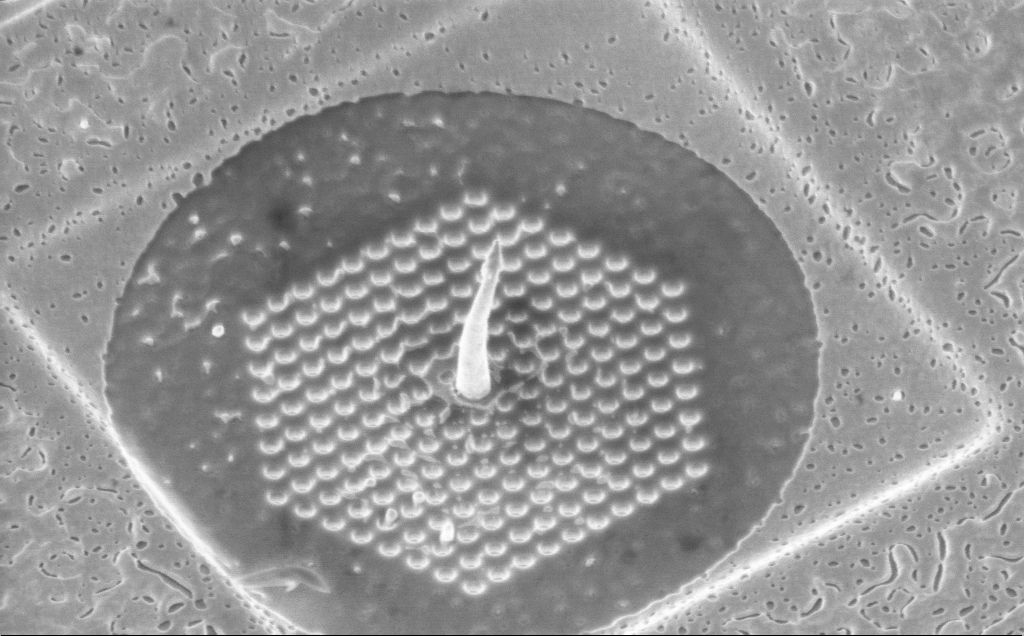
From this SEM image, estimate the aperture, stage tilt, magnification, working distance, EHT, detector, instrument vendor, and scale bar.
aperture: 30 µm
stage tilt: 34.3°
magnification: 43.26 K X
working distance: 6 mm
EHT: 3 kV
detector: InLens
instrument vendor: Zeiss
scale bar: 1000 nm